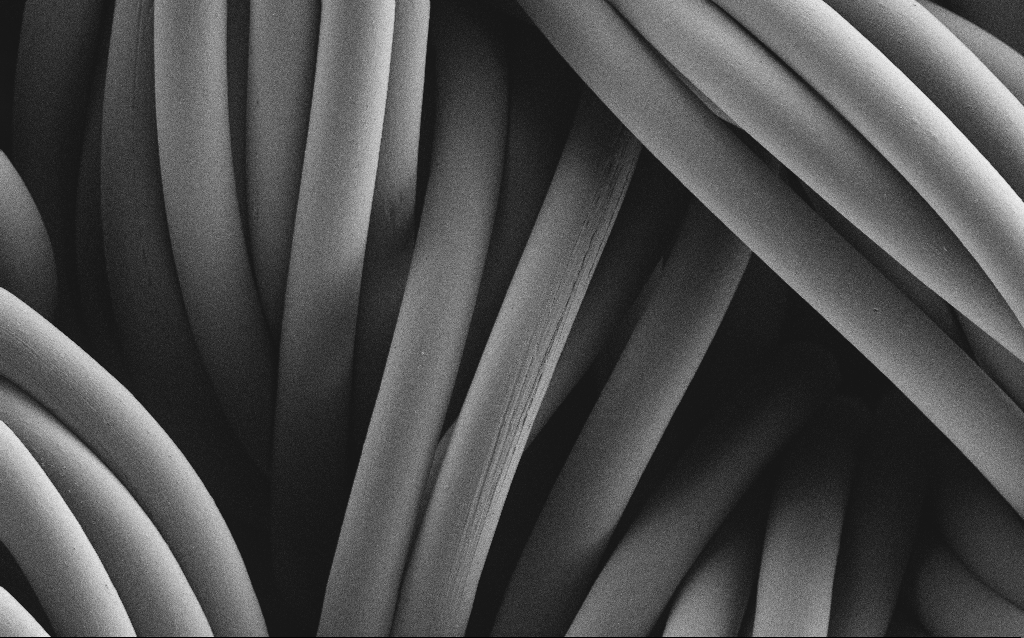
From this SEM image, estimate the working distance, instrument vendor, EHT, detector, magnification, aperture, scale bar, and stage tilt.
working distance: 4 mm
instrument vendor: Zeiss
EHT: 1 kV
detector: SE2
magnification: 1.14 K X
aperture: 30 µm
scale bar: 20000 nm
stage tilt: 0°